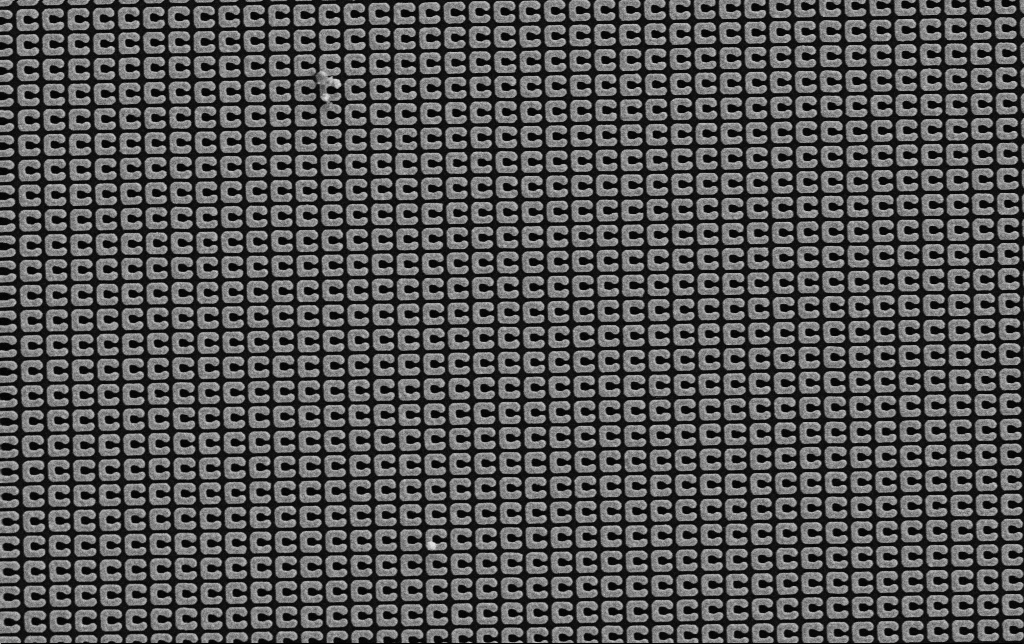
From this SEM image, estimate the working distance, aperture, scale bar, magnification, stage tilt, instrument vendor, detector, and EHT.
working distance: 4.3 mm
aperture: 30 µm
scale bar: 2000 nm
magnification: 20.06 K X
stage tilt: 0°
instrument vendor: Zeiss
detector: SE2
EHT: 5 kV